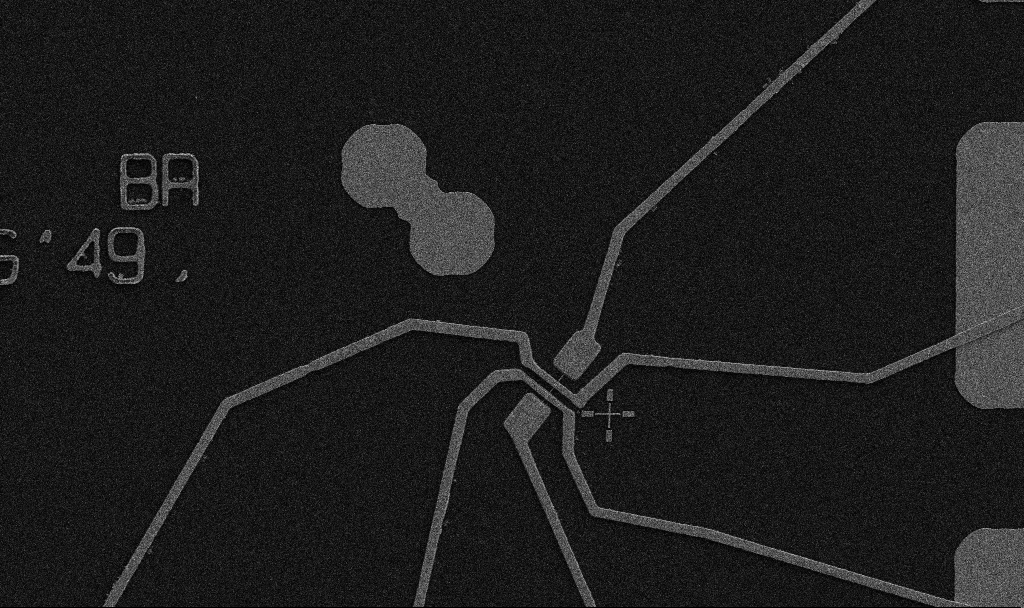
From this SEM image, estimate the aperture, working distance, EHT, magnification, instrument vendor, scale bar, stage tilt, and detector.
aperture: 30 µm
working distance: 10.7 mm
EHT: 5 kV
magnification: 5 K X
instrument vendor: Zeiss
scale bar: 10000 nm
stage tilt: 0°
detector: SE2